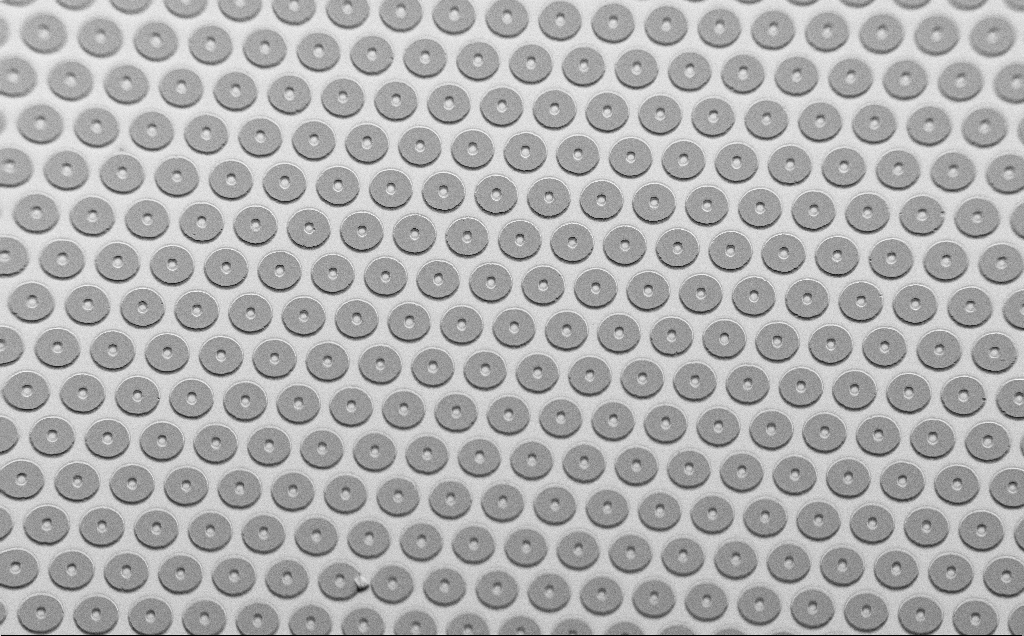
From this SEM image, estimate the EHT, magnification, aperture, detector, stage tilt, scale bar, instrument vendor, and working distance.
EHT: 1.5 kV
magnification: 0.323 K X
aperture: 30 µm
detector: SE2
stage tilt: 45°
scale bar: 200000 nm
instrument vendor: Zeiss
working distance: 7 mm